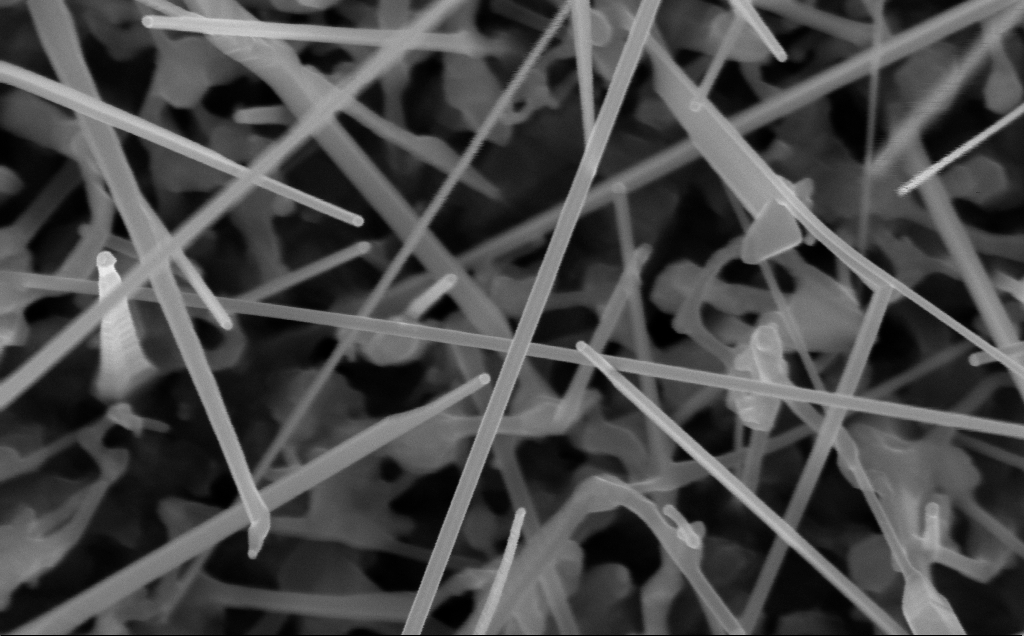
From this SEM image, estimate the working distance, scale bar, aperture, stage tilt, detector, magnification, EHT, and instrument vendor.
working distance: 8 mm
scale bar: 200 nm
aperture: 30 µm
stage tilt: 0°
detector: InLens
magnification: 135.94 K X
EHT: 10 kV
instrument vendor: Zeiss